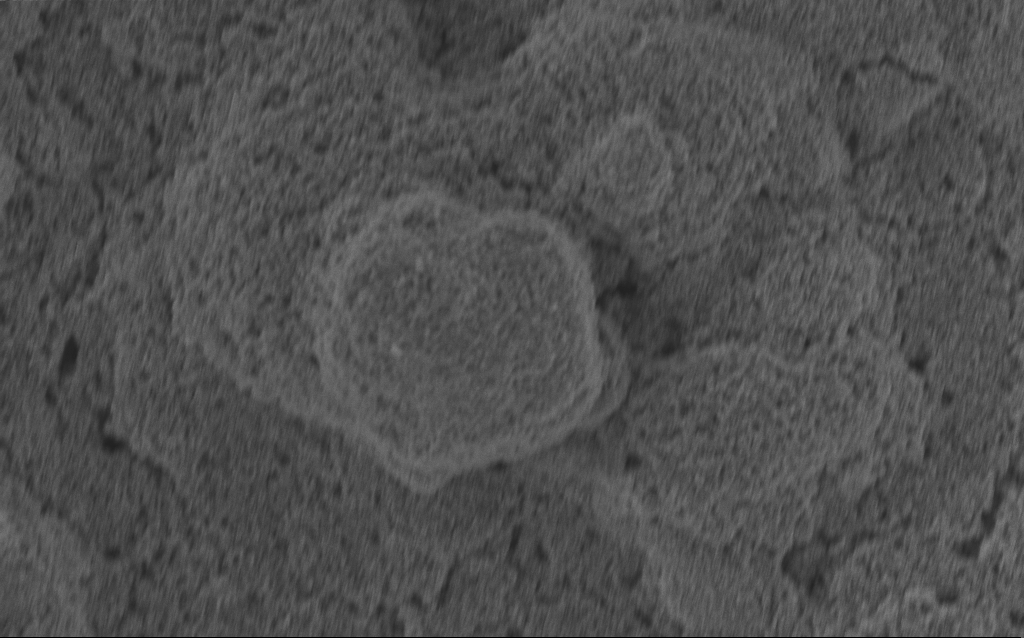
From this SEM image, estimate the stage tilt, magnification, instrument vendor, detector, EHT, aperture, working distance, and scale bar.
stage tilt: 0°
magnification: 15.33 K X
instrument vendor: Zeiss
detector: InLens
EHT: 3 kV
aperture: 20 µm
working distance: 2.6 mm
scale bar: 1000 nm